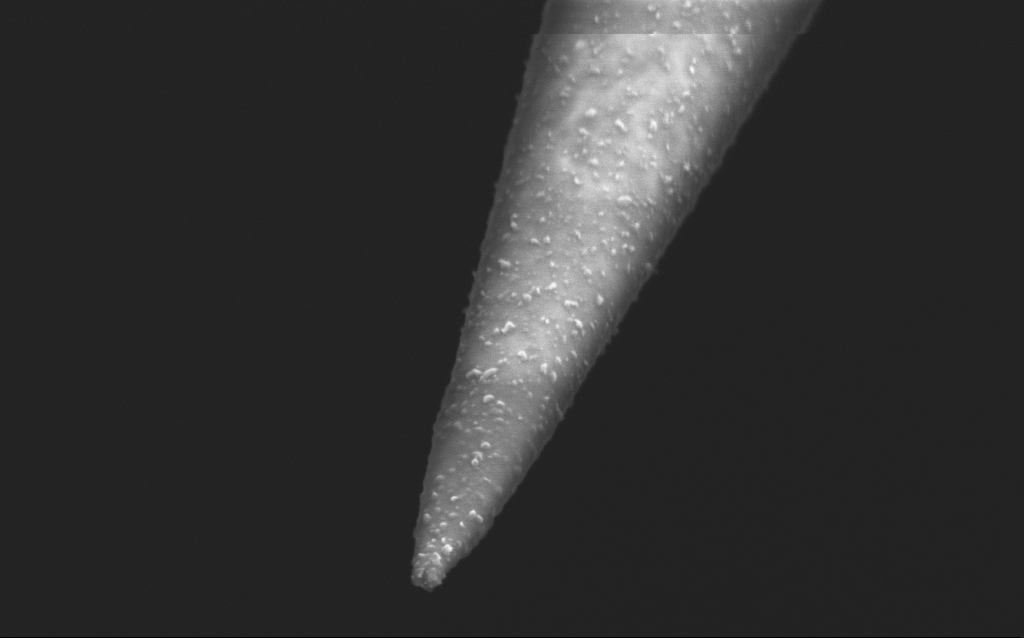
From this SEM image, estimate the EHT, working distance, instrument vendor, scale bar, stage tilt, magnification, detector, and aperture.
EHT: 2.5 kV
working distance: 5 mm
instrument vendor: Zeiss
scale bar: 200 nm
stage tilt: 45°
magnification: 100 K X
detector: InLens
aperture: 30 µm